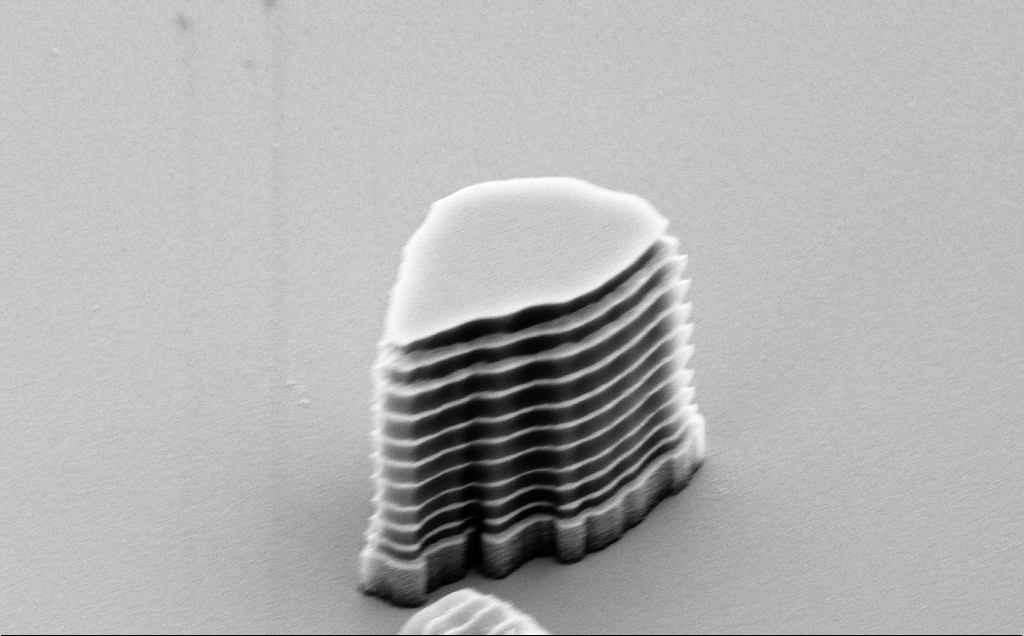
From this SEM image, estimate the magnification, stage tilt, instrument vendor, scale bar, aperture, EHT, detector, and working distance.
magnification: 50.42 K X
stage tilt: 50°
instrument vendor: Zeiss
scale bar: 1000 nm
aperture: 30 µm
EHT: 5 kV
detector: SE2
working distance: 10 mm